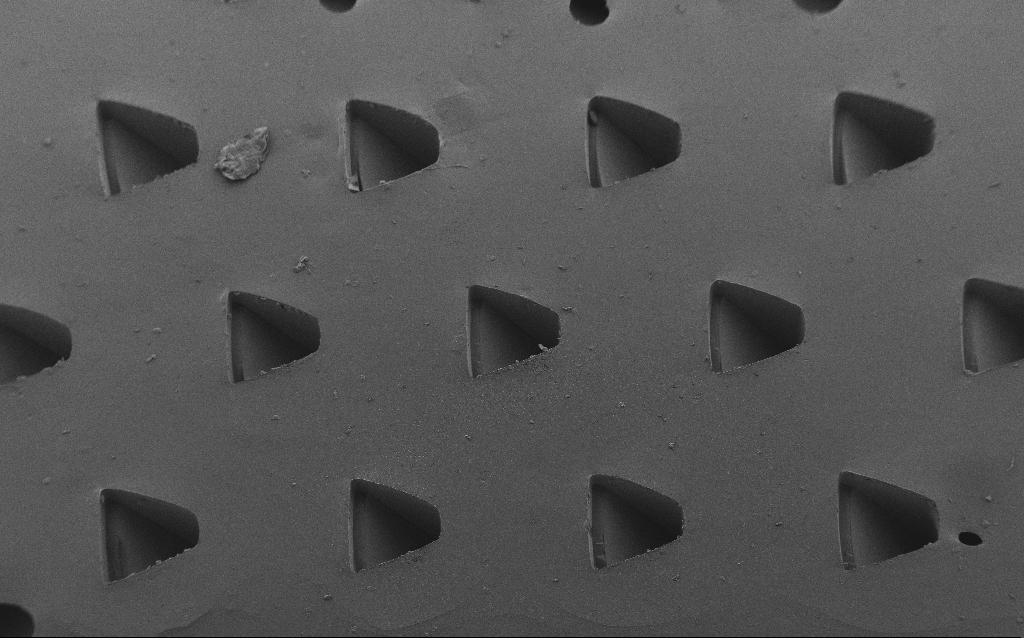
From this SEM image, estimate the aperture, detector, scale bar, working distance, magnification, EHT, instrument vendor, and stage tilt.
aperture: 30 µm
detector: SE2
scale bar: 200000 nm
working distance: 7 mm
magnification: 0.09 K X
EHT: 5 kV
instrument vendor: Zeiss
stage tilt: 35°